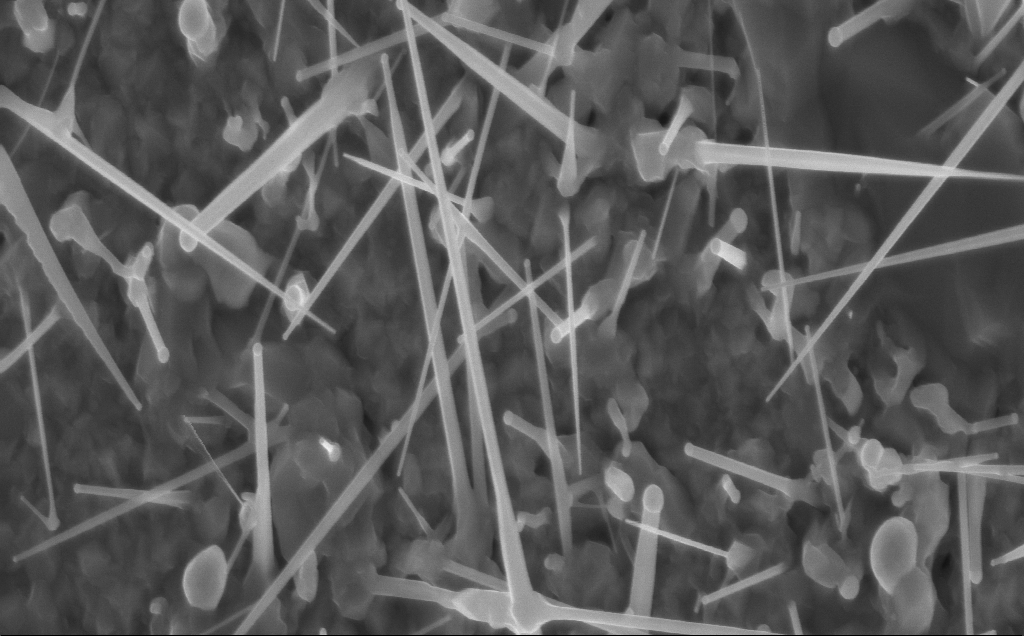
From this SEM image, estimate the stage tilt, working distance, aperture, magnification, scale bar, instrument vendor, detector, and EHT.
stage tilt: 0°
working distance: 4 mm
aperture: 30 µm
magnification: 40 K X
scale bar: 1000 nm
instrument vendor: Zeiss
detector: InLens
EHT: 10 kV